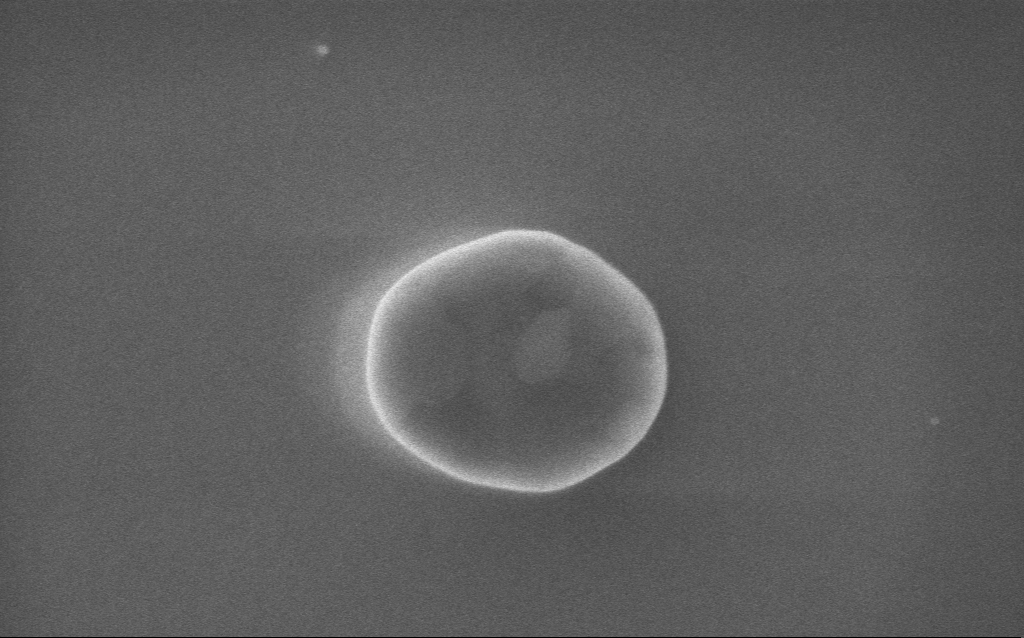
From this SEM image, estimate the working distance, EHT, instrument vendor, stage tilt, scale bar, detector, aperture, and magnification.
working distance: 2 mm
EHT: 5 kV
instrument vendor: Zeiss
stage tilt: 0°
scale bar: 200 nm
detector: InLens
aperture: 30 µm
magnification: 116.62 K X